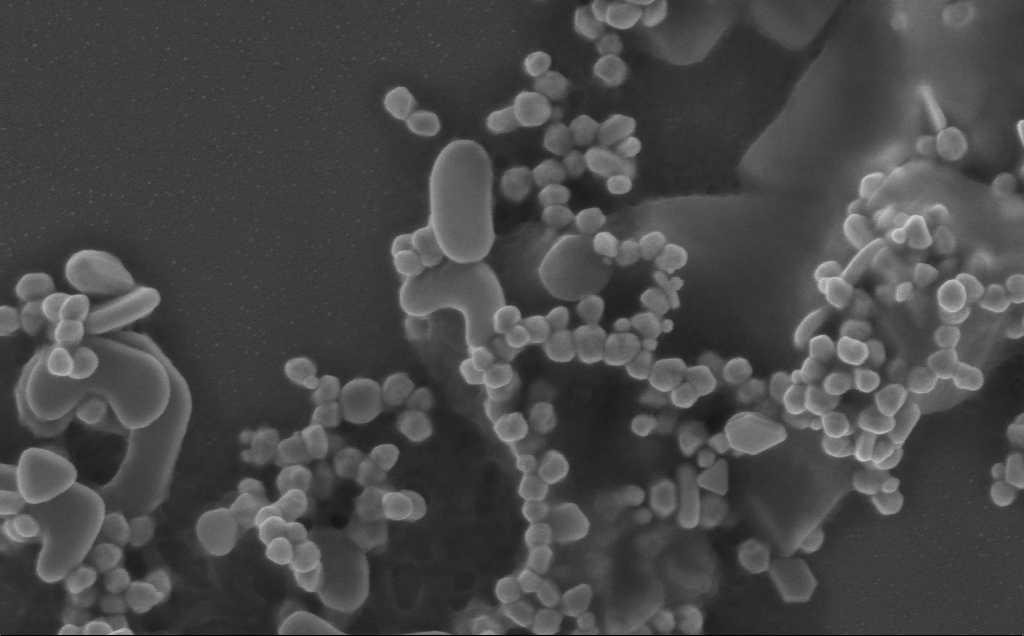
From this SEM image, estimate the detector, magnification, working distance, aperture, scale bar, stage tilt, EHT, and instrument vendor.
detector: InLens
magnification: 80 K X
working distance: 3 mm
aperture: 30 µm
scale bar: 200 nm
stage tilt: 0°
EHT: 5 kV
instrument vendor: Zeiss